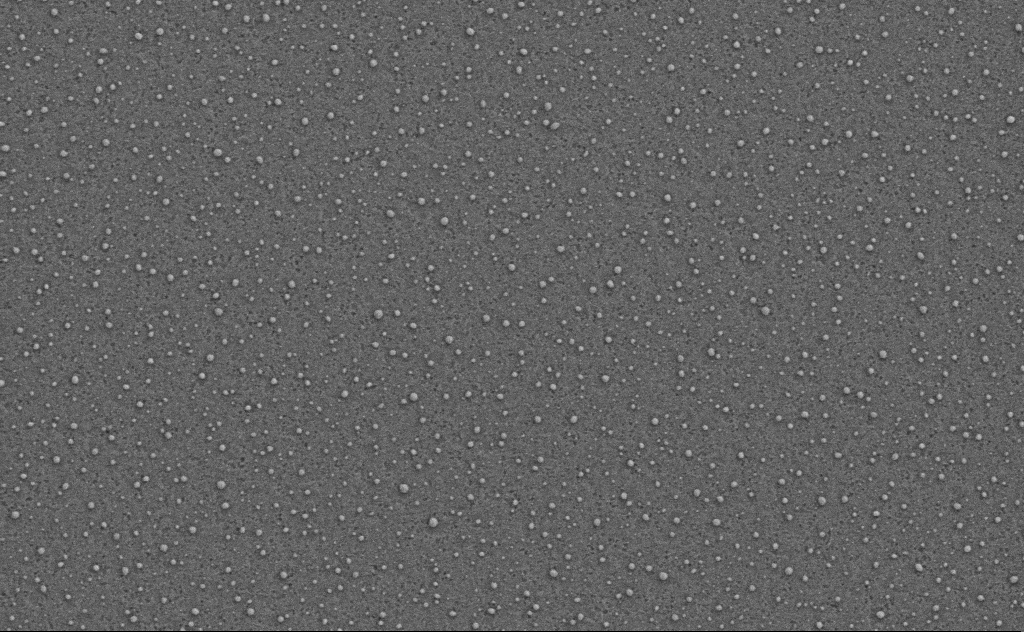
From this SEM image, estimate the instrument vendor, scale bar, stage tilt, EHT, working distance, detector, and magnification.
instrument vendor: Zeiss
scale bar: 1000 nm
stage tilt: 0°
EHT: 3 kV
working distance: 4 mm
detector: SE2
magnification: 40 K X